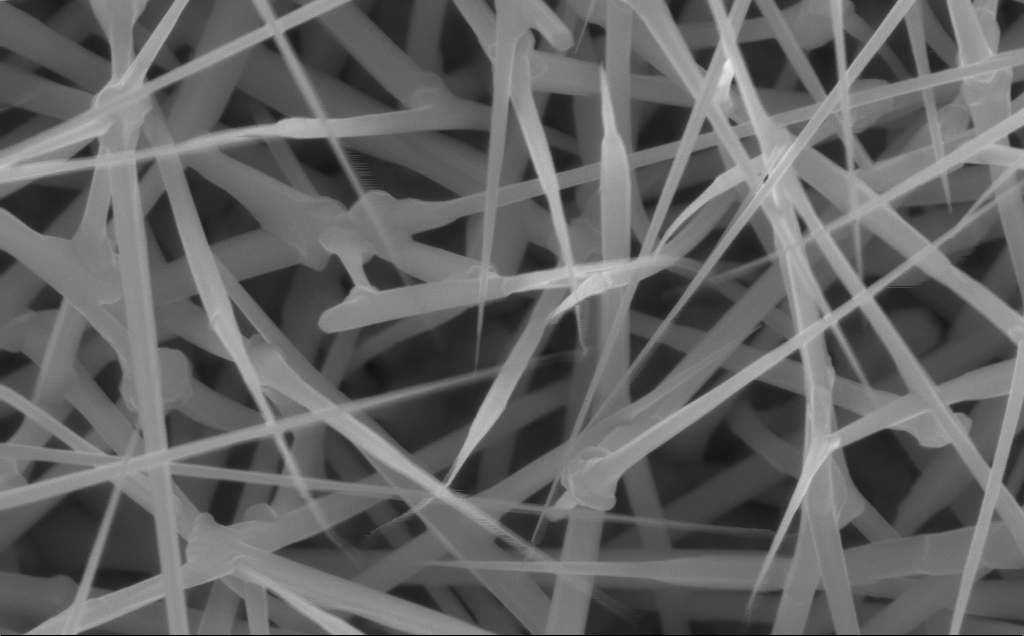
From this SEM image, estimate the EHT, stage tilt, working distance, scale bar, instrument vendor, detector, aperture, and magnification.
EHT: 10 kV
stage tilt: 0°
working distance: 6 mm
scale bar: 200 nm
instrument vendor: Zeiss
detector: InLens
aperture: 30 µm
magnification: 80 K X